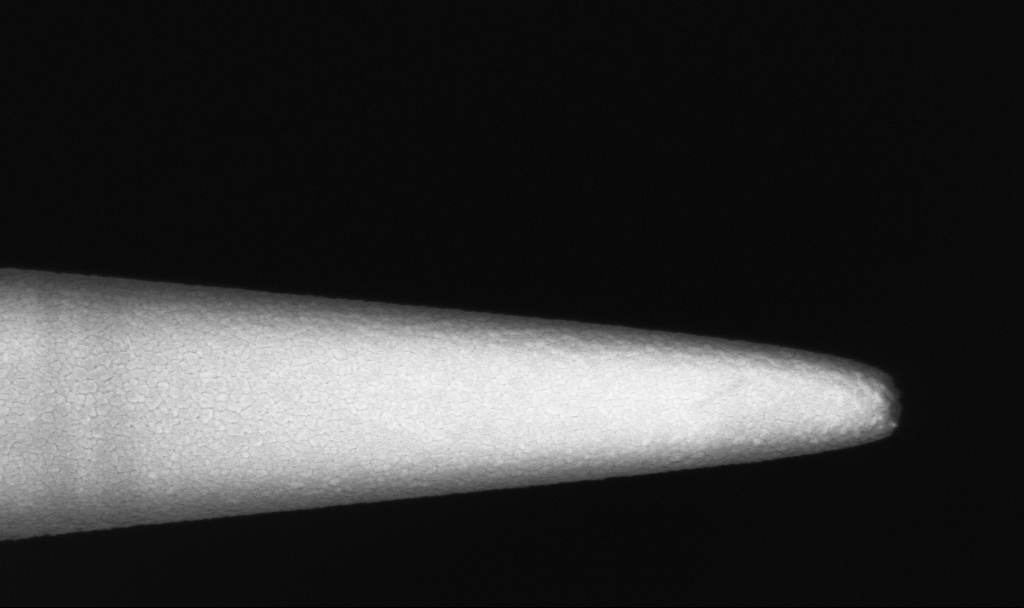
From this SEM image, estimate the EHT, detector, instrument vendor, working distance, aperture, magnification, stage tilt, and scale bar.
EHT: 1.5 kV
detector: InLens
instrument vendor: Zeiss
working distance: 3.6 mm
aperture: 30 µm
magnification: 50 K X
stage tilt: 0°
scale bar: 1000 nm